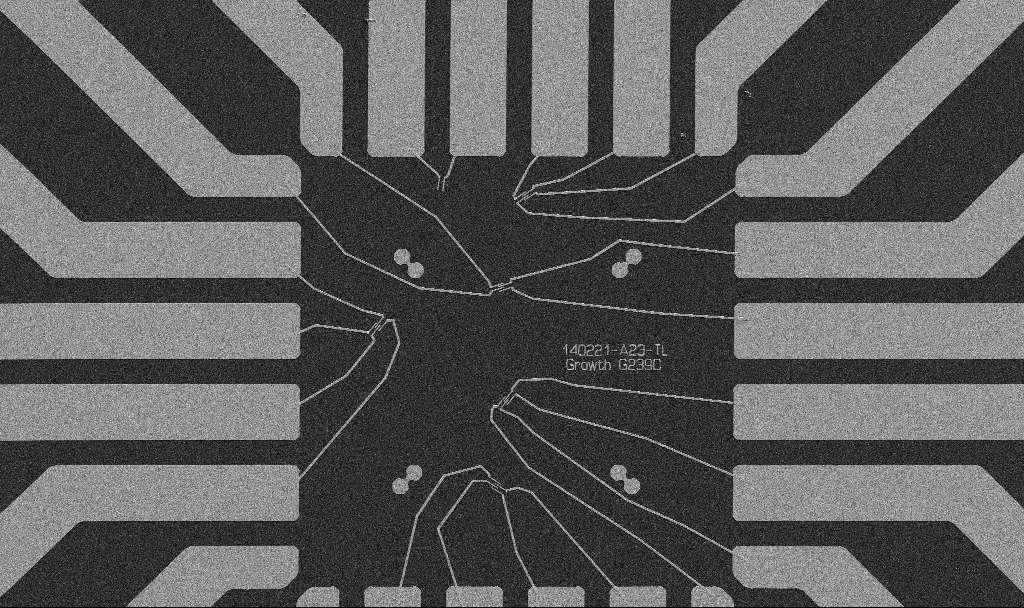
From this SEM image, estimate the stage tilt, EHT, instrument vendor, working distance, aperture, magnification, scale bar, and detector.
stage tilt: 0°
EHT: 5 kV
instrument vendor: Zeiss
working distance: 10.7 mm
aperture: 30 µm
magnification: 1 K X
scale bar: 20000 nm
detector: SE2